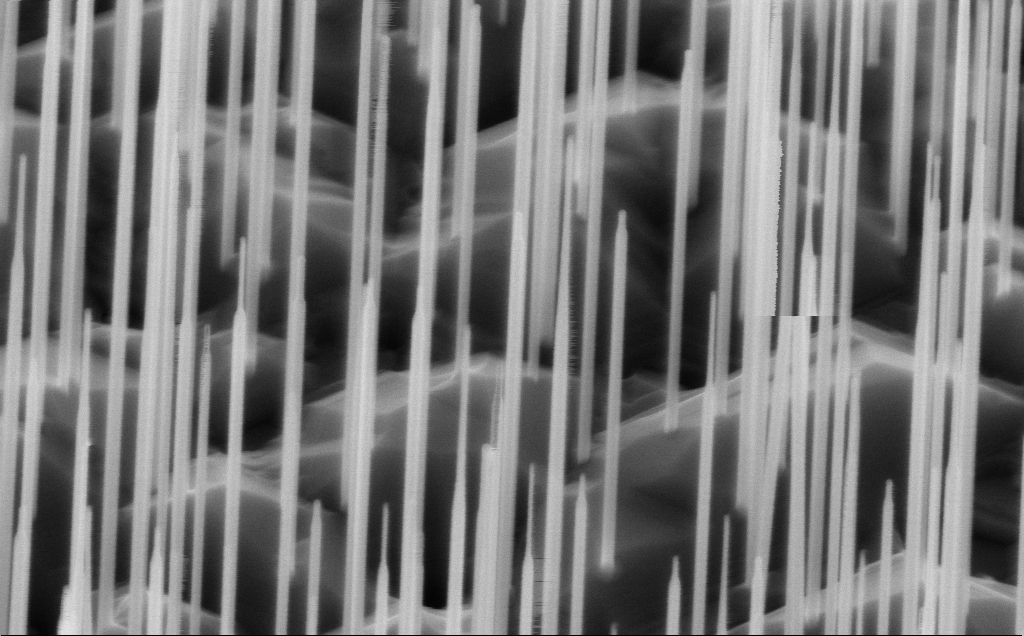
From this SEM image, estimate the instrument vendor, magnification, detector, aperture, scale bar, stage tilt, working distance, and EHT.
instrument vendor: Zeiss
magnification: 80 K X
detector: InLens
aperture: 30 µm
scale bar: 200 nm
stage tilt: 45°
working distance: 5 mm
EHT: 10 kV